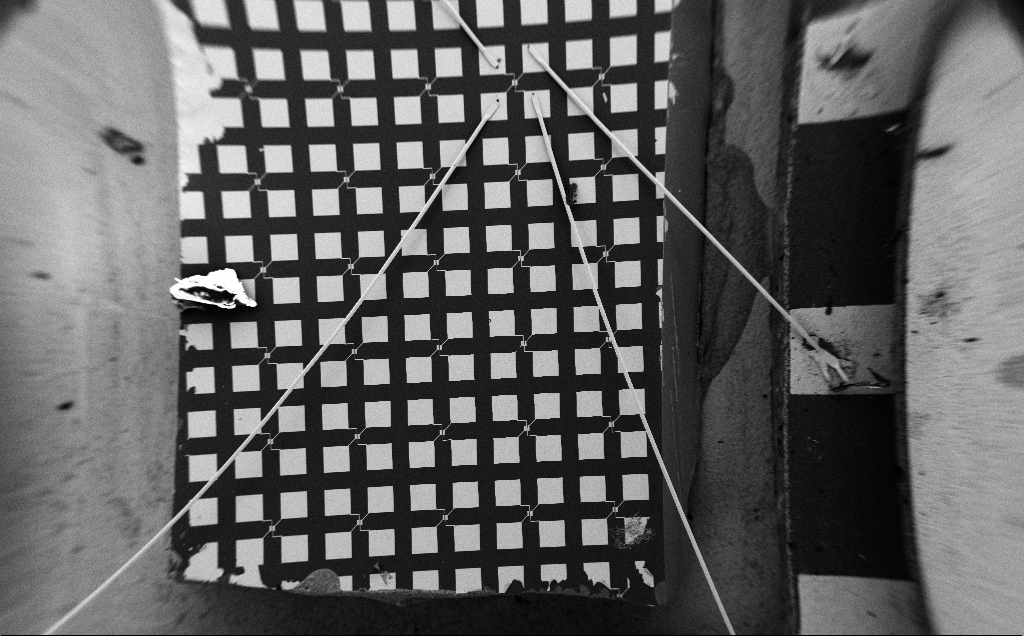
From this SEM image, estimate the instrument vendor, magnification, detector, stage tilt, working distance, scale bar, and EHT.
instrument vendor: Zeiss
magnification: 0.064 K X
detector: SE2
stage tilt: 0°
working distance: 10 mm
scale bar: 1e+06 nm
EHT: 5 kV